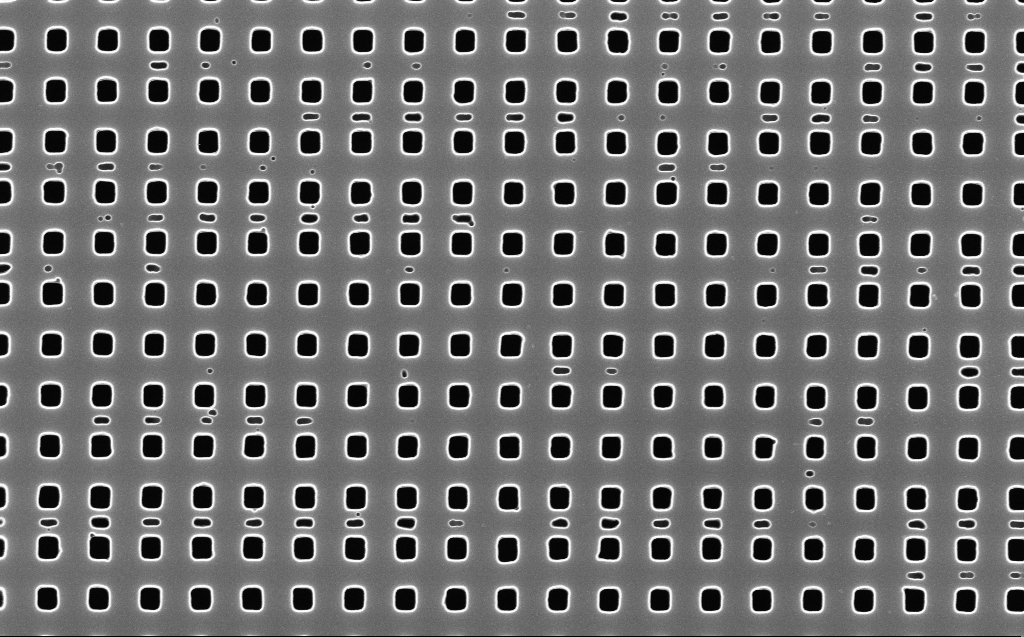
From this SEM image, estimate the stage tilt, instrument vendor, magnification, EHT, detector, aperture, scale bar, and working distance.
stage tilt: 0°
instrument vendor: Zeiss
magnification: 37.76 K X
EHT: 10 kV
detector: InLens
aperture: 30 µm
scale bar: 1000 nm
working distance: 6 mm